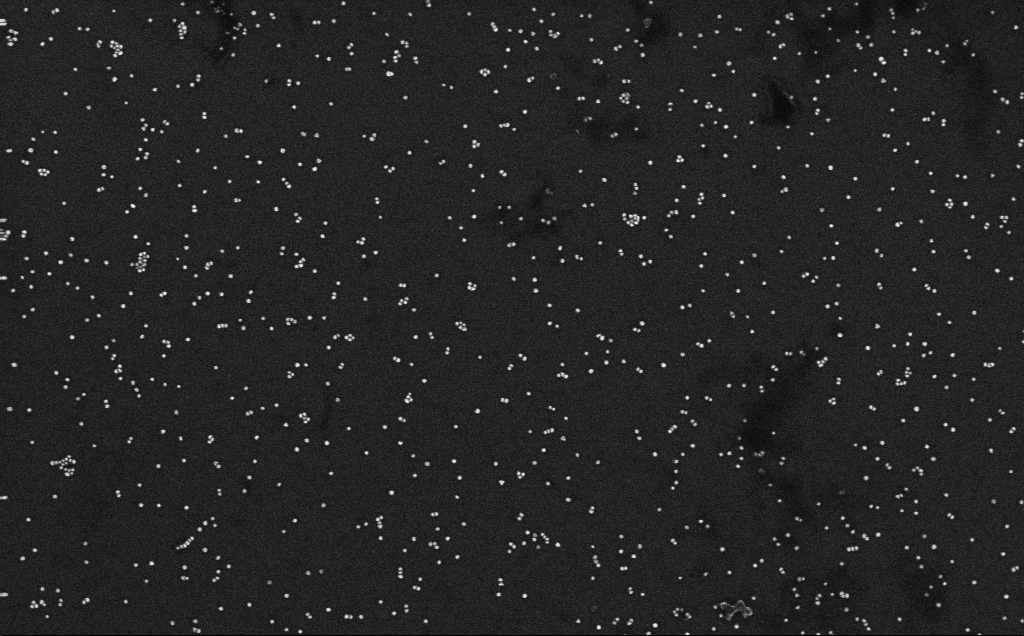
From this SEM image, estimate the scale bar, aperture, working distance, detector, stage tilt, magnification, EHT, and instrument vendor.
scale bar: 200 nm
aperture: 30 µm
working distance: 3.3 mm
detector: InLens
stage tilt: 0°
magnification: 100 K X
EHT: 10 kV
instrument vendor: Zeiss